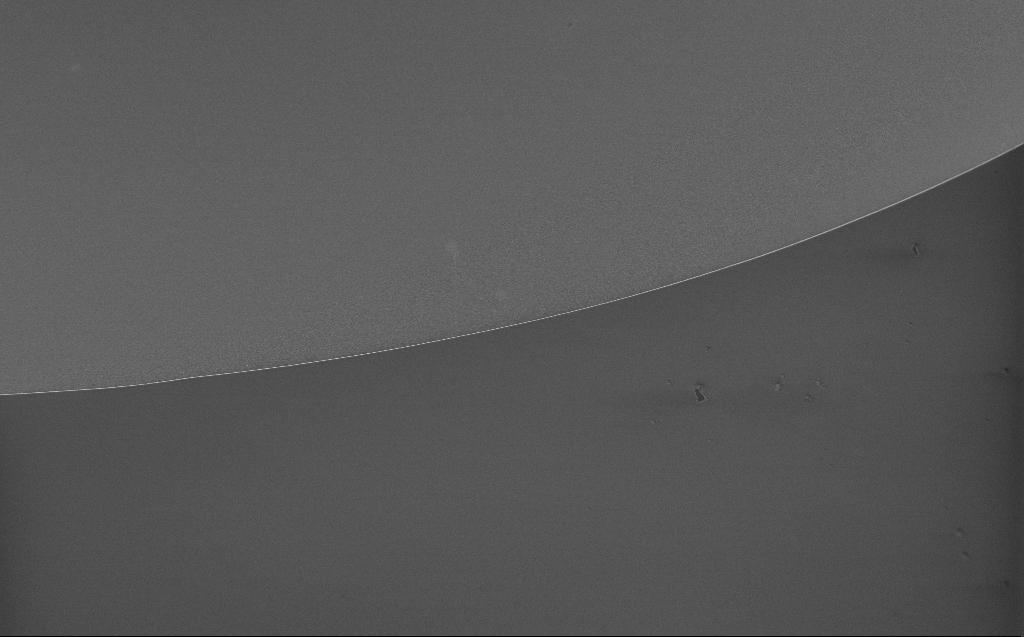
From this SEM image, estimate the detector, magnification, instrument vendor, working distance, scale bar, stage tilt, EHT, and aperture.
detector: InLens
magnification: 1.97 K X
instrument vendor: Zeiss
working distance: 6 mm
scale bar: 20000 nm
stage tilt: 44.9°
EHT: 1.5 kV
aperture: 20 µm